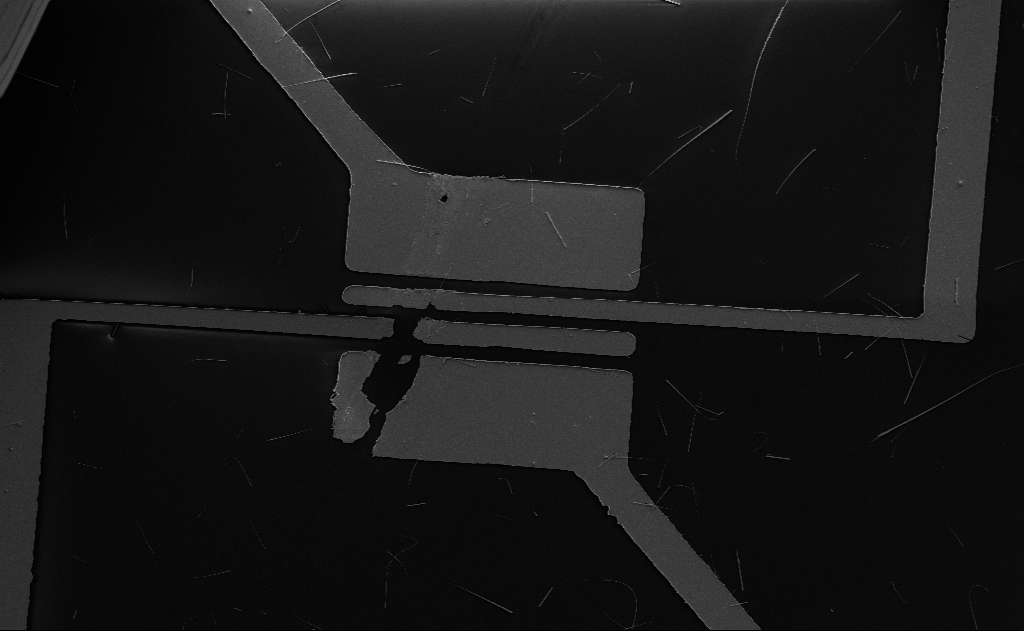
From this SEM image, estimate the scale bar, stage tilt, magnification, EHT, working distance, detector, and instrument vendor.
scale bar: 10000 nm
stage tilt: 0°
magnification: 3.56 K X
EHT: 5 kV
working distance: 15 mm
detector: SE2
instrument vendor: Zeiss